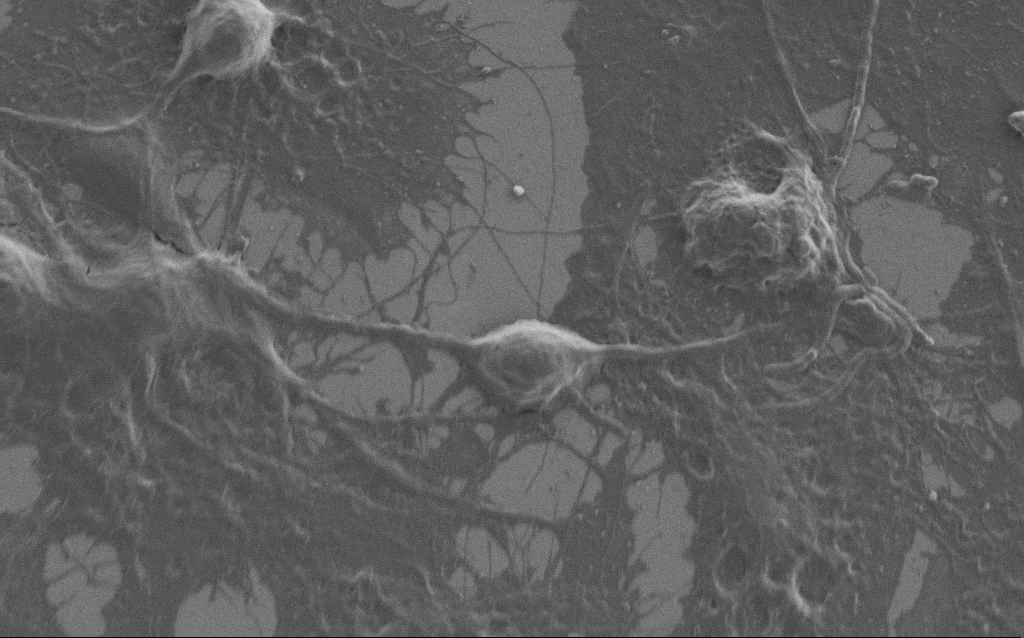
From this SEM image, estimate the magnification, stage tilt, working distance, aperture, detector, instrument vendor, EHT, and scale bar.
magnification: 5 K X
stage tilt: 0°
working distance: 6 mm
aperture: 30 µm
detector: SE2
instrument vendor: Zeiss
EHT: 1 kV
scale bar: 10000 nm